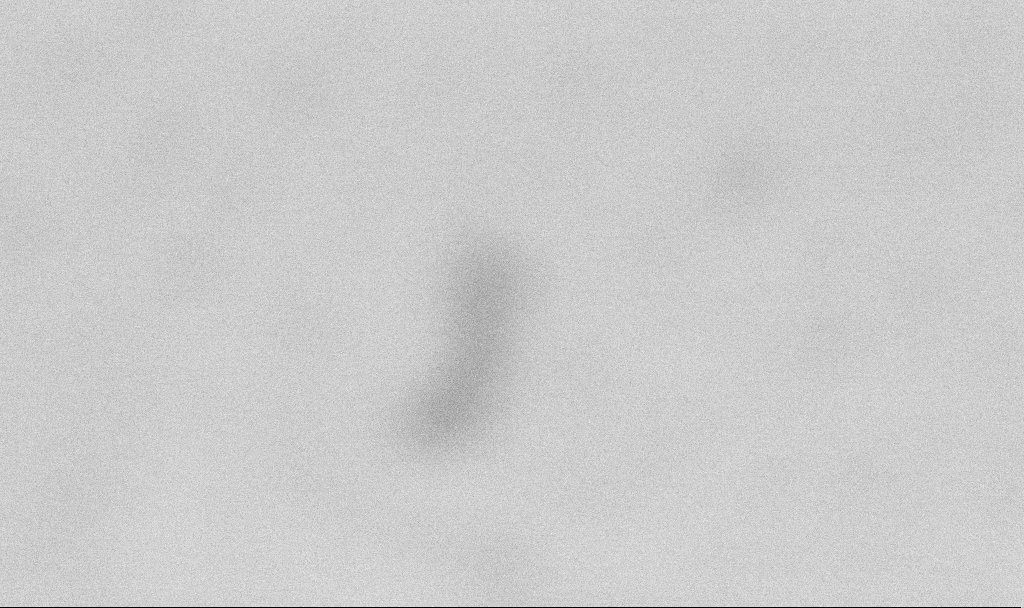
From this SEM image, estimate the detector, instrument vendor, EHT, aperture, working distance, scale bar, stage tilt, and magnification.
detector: SE2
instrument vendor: Zeiss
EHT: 2 kV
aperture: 30 µm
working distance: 6.5 mm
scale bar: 100 nm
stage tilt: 0°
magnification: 500 K X